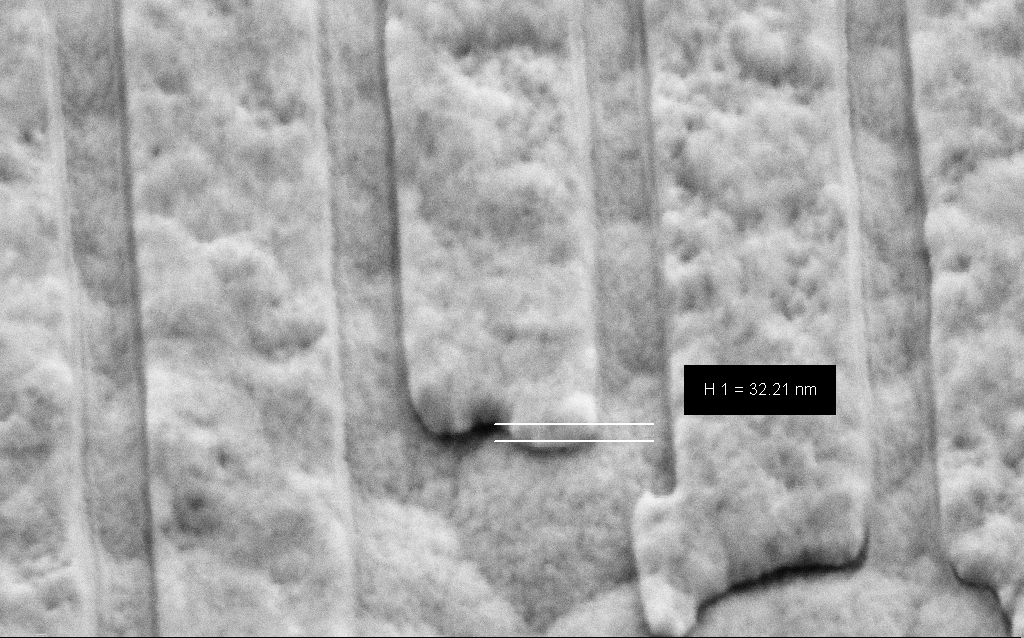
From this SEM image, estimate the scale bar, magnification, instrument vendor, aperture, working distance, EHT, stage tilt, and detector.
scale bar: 200 nm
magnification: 193.81 K X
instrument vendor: Zeiss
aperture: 30 µm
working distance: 6 mm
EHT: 3 kV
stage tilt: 45°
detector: SE2